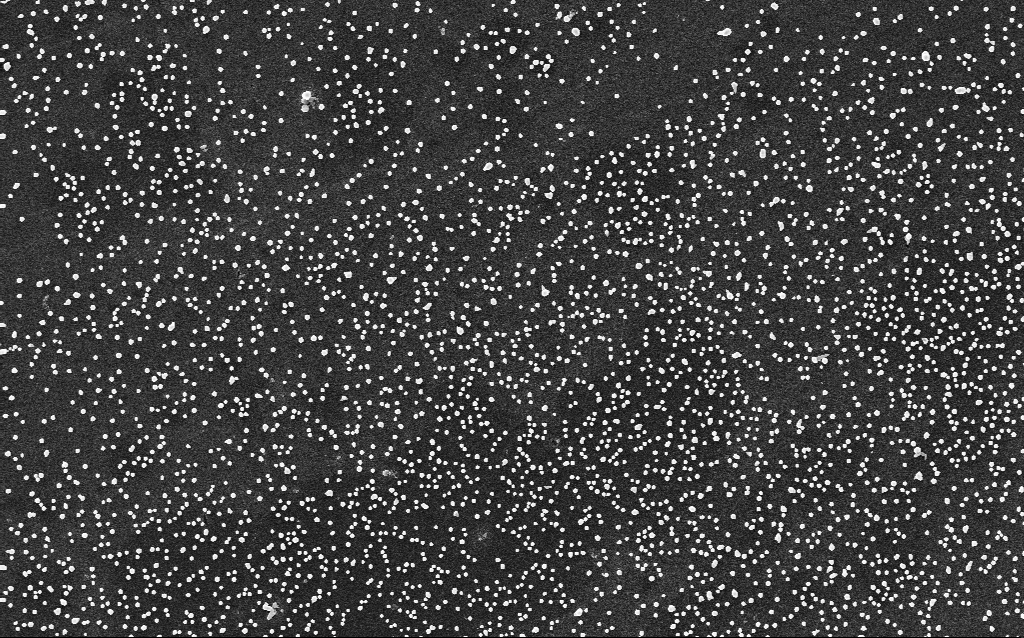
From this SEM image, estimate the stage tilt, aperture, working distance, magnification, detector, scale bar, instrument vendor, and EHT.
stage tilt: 0°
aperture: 30 µm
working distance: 2.8 mm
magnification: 10 K X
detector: InLens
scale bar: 2000 nm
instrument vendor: Zeiss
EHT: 5 kV